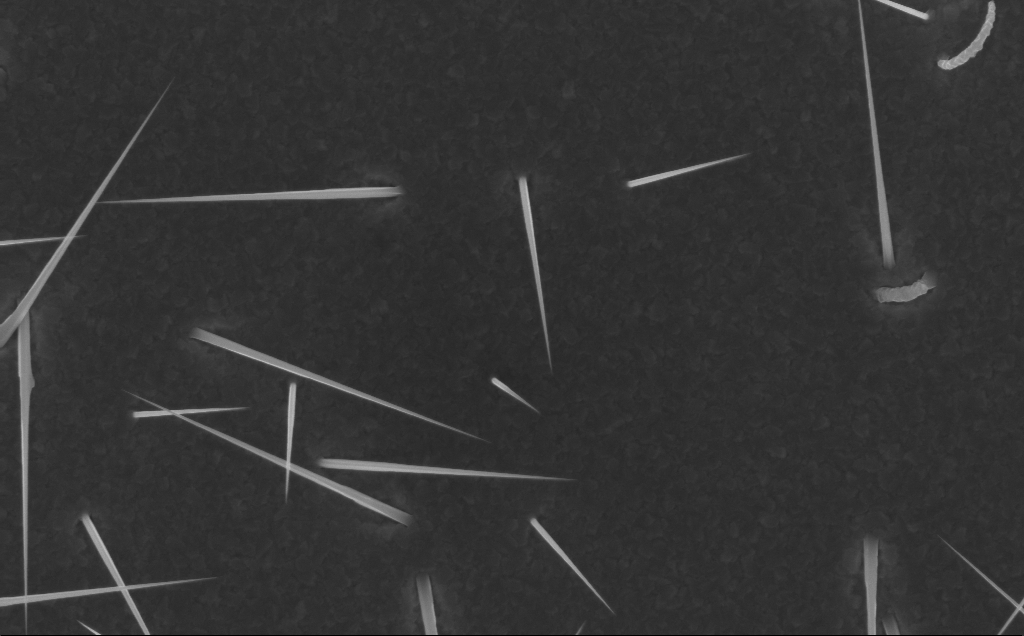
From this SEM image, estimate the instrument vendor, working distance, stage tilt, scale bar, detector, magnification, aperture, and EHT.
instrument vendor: Zeiss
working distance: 5 mm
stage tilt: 0°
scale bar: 1000 nm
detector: InLens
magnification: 20 K X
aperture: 30 µm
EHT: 10 kV